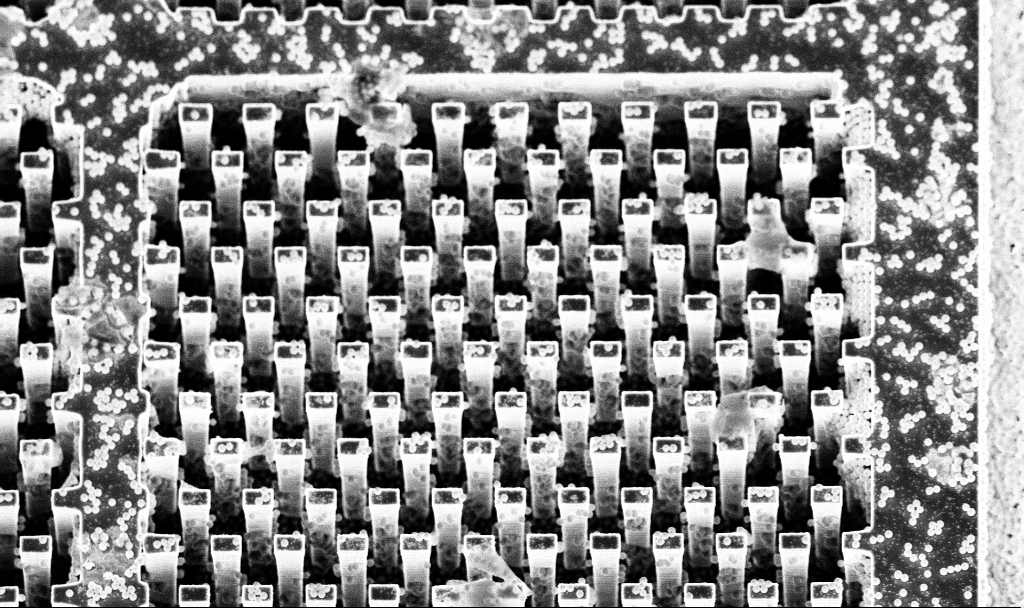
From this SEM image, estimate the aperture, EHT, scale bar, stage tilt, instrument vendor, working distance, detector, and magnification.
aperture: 30 µm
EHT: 5 kV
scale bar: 10000 nm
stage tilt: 20°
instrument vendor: Zeiss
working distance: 4.6 mm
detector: InLens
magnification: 4.78 K X